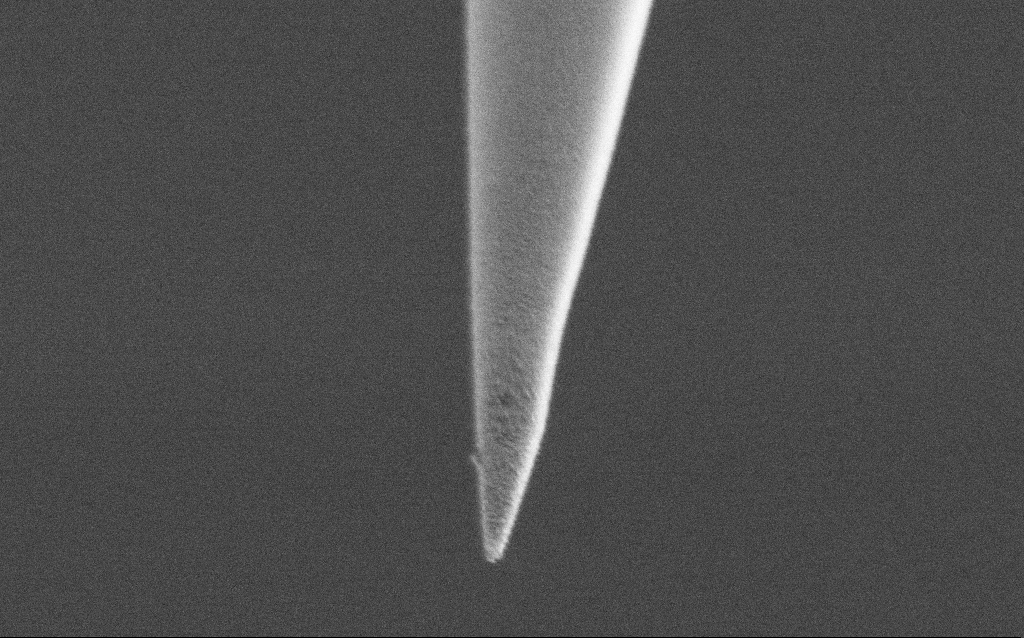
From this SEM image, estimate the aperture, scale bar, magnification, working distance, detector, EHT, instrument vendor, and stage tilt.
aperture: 30 µm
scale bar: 1000 nm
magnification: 50 K X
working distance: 6.9 mm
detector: SE2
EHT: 1 kV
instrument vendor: Zeiss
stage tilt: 45°